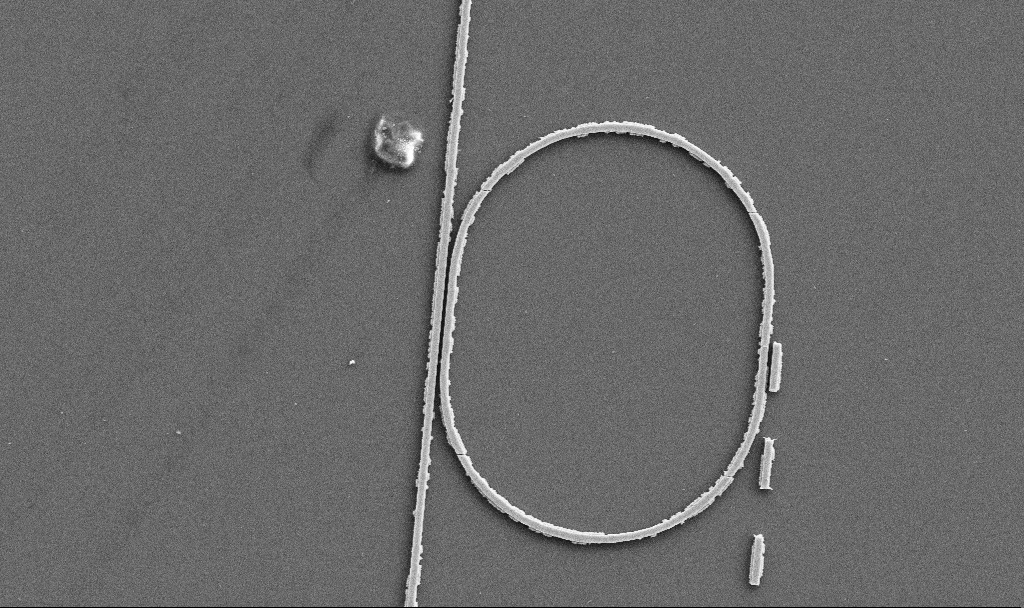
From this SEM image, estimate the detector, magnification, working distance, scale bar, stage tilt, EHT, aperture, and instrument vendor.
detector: SE2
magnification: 5.99 K X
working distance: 7.4 mm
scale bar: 10000 nm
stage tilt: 0°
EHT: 5 kV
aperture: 30 µm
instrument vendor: Zeiss